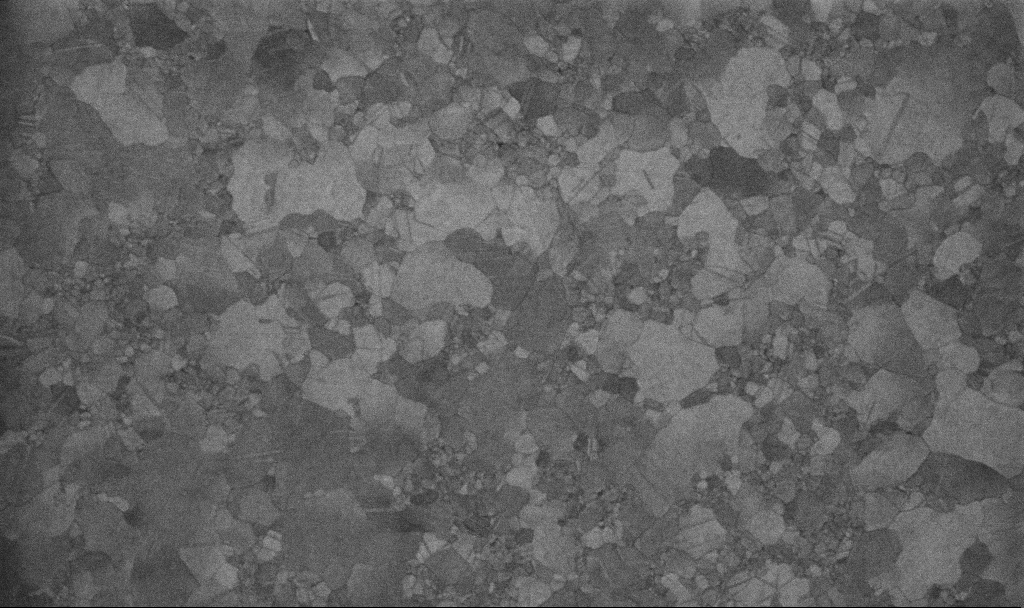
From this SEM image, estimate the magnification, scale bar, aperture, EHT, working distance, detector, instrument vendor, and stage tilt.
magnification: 100 K X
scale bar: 200 nm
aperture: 30 µm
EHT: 10 kV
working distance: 3.4 mm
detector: InLens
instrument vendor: Zeiss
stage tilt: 0°